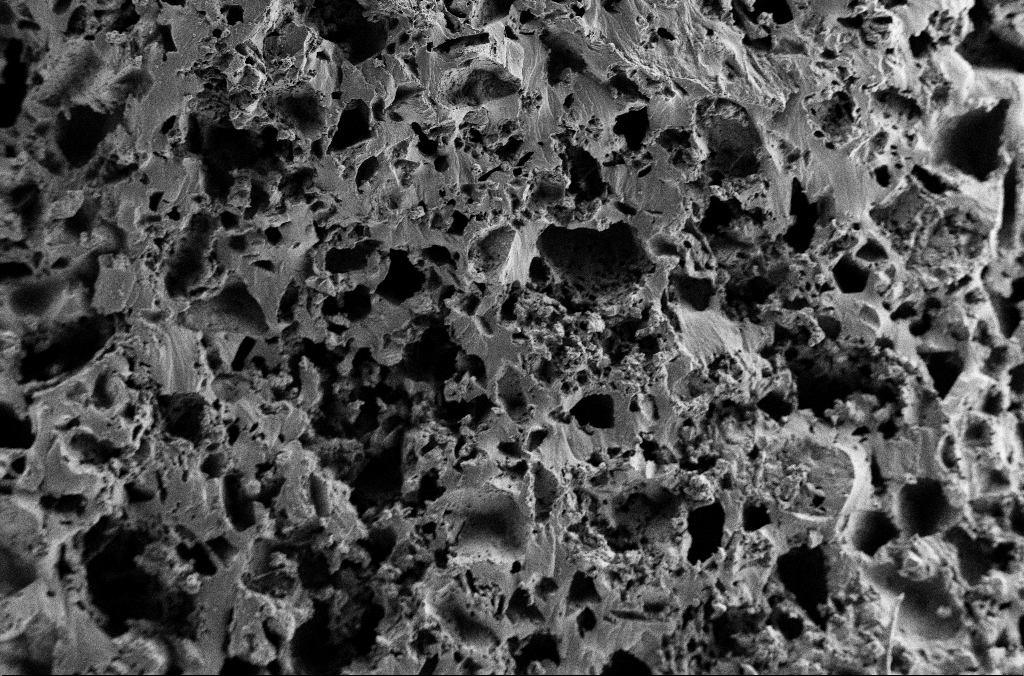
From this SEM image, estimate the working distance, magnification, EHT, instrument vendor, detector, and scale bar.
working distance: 3 mm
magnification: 0.25 K X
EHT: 2 kV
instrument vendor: Zeiss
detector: SE2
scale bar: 100000 nm